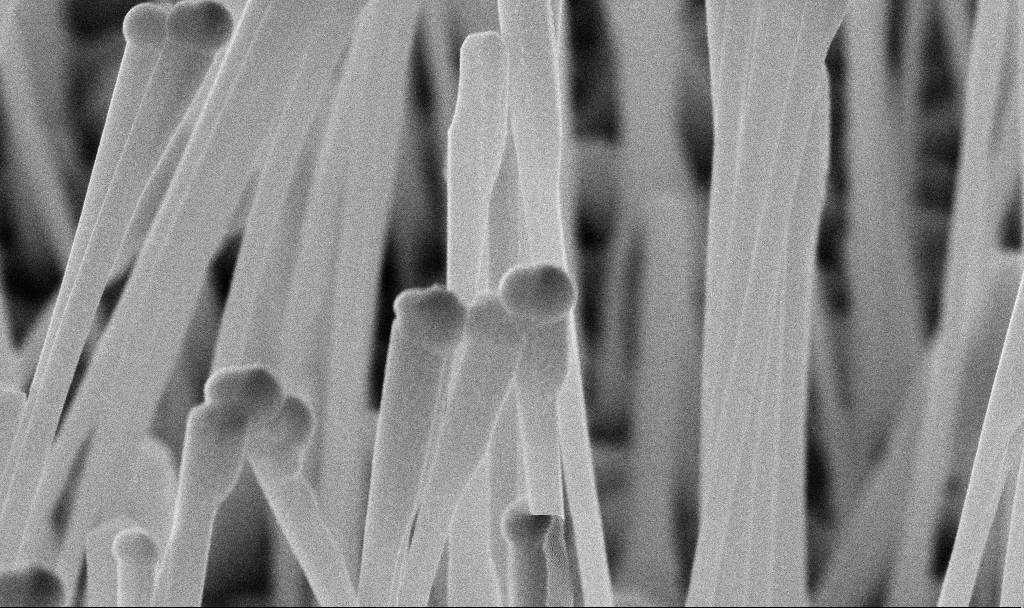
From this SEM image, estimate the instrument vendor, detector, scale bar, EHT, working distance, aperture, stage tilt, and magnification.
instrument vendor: Zeiss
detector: InLens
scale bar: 200 nm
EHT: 10 kV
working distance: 7 mm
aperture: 30 µm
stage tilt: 45°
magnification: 100 K X